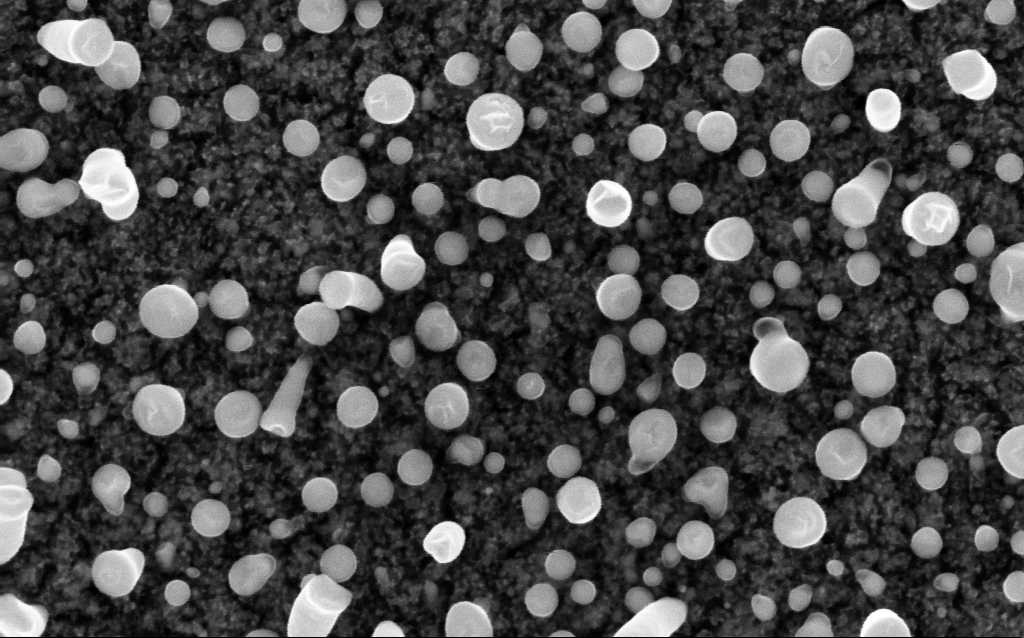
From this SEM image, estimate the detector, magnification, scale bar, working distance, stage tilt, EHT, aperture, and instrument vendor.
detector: InLens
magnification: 200 K X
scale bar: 100 nm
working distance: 2.1 mm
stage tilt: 0°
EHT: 5 kV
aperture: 30 µm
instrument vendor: Zeiss